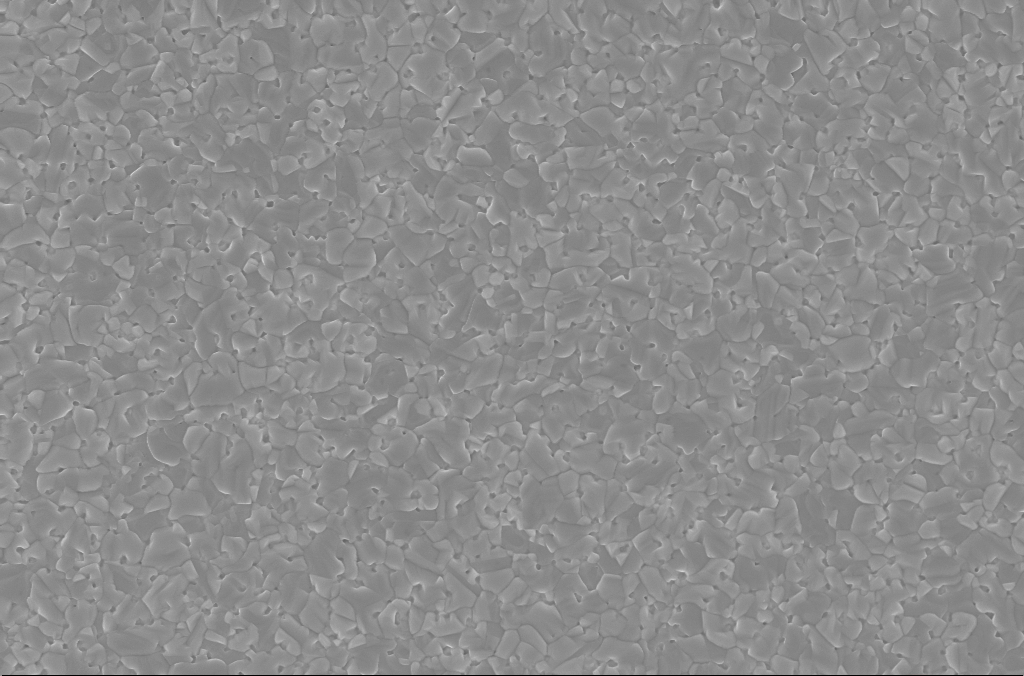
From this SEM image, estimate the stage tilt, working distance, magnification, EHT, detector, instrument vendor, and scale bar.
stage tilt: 0°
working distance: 3 mm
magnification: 20 K X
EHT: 5 kV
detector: InLens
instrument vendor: Zeiss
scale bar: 2000 nm